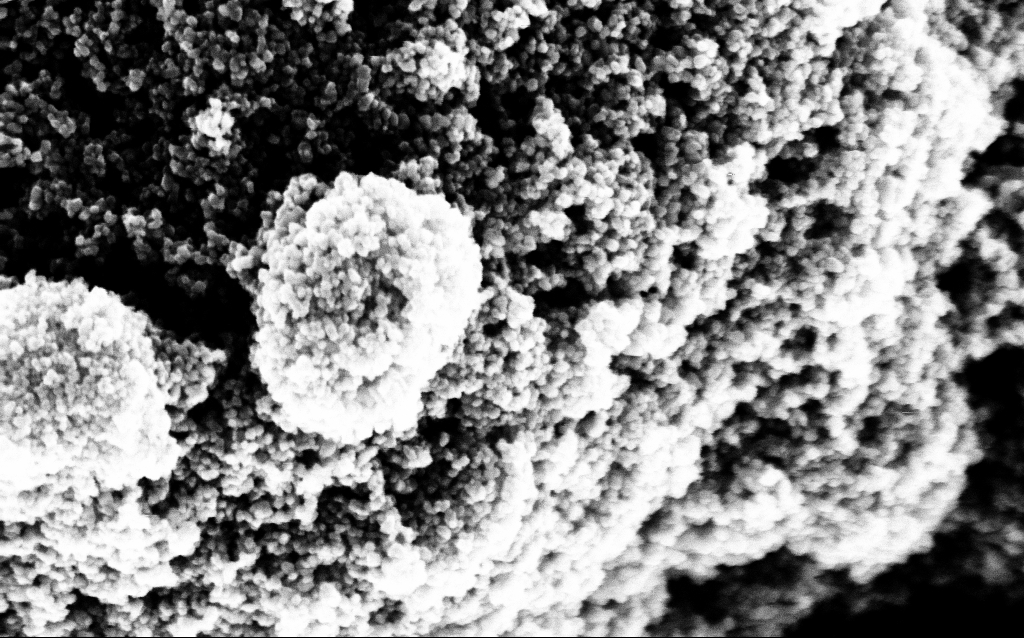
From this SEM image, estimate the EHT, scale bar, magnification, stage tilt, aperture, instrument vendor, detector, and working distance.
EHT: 5 kV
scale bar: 100 nm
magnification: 135.03 K X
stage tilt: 0°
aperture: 30 µm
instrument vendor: Zeiss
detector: InLens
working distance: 1.8 mm